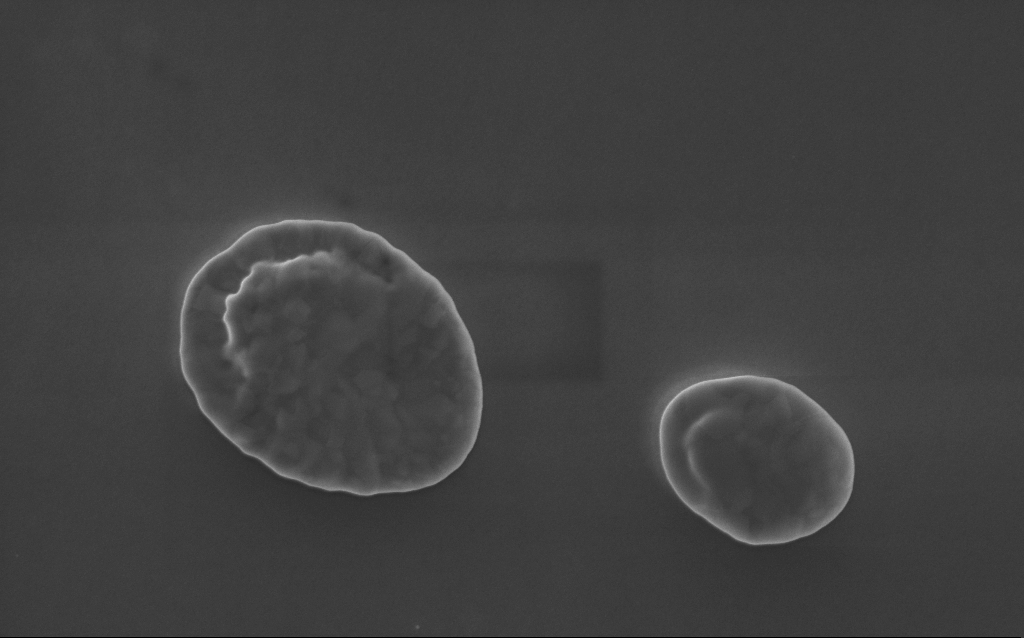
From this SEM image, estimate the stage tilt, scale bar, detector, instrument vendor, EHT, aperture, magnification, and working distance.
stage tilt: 0°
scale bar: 1000 nm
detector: InLens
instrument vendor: Zeiss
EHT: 5 kV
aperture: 30 µm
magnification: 58 K X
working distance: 3 mm